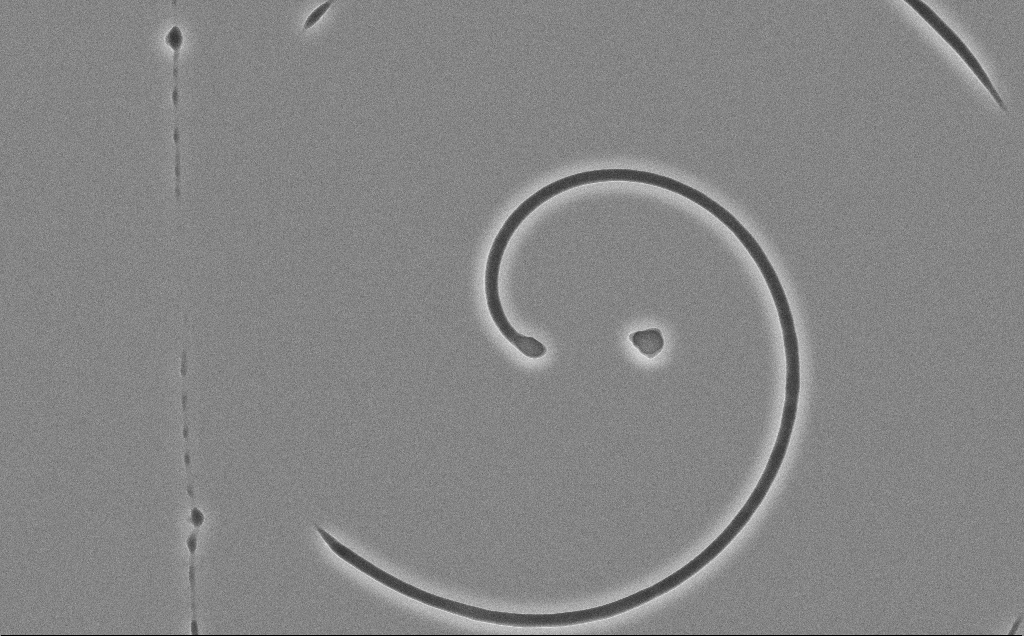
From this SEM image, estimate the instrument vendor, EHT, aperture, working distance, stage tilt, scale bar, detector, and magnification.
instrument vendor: Zeiss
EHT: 10 kV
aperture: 30 µm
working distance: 12 mm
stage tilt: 0°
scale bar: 10000 nm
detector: SE2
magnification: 4.09 K X